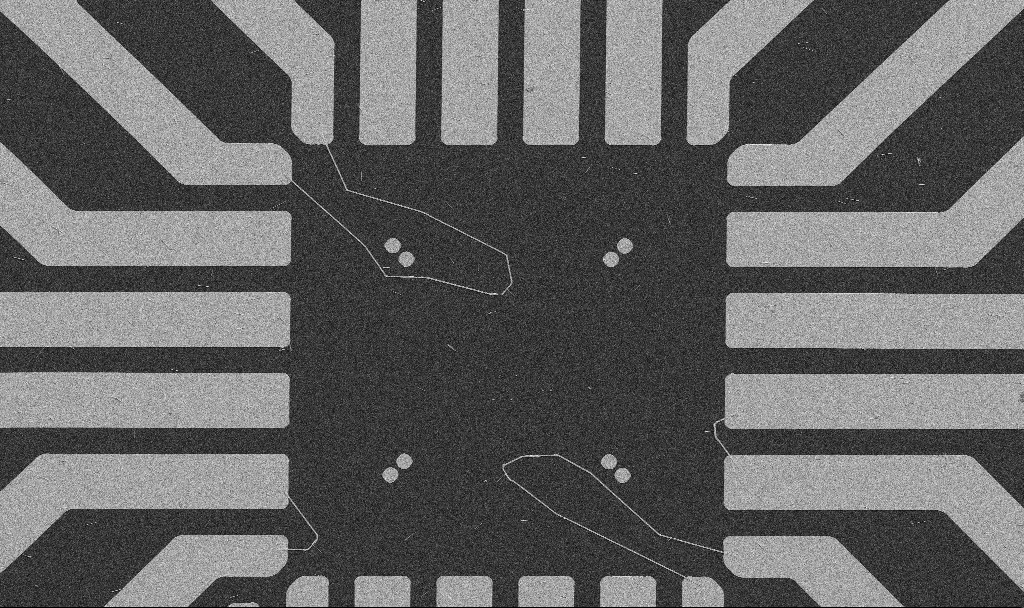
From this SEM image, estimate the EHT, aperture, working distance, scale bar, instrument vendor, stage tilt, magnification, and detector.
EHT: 5 kV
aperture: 30 µm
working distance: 10.7 mm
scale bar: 20000 nm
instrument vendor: Zeiss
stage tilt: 0°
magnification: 1 K X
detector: SE2